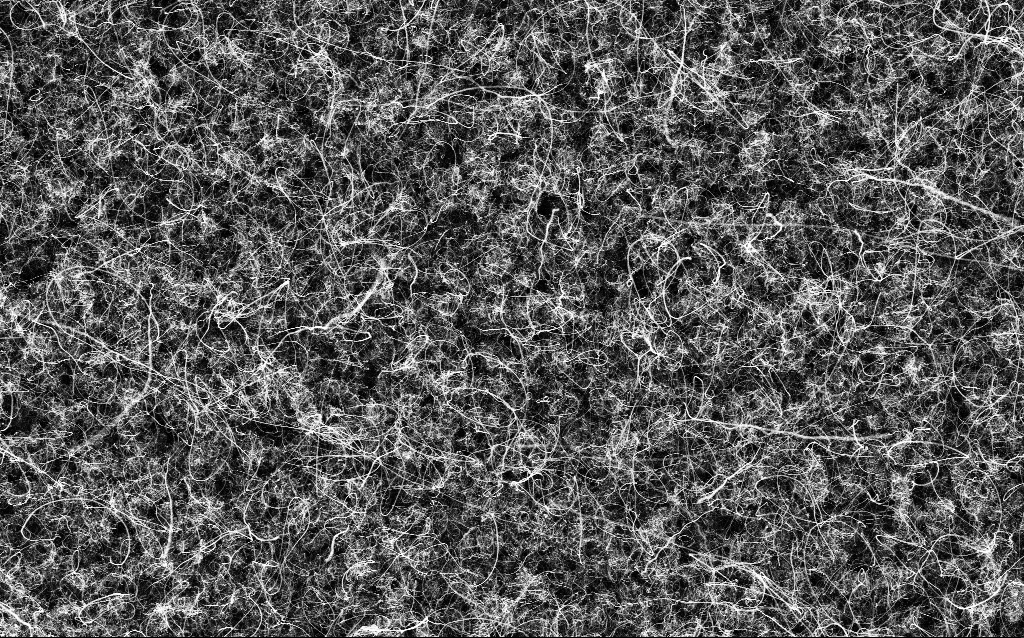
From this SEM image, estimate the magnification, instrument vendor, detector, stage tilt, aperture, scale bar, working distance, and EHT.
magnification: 10 K X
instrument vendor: Zeiss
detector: InLens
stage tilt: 0°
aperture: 30 µm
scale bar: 2000 nm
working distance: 3.6 mm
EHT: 5 kV